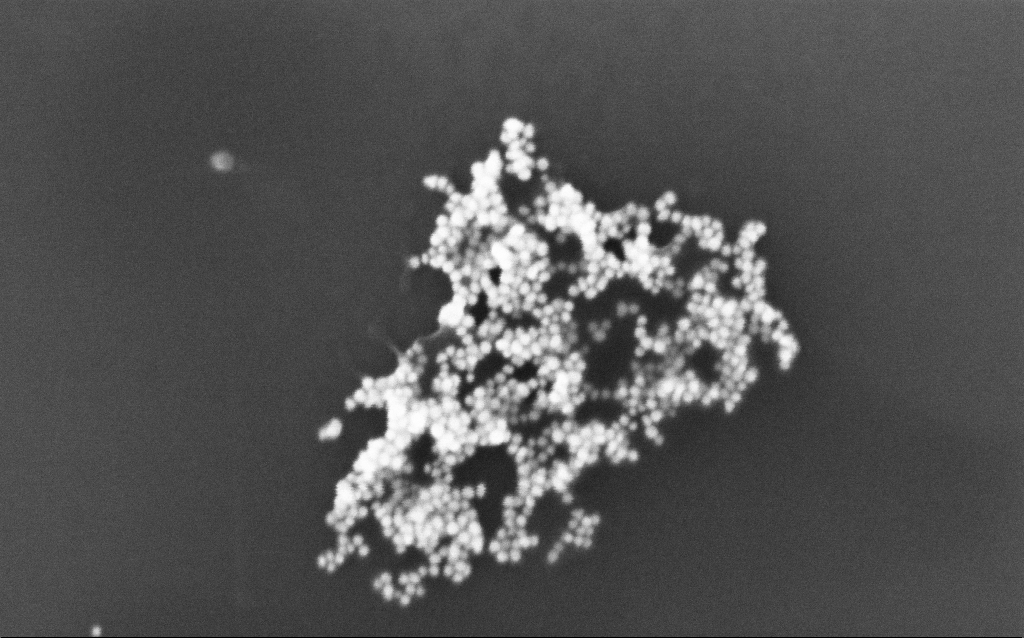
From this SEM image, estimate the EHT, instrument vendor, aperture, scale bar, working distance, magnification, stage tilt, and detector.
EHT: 4 kV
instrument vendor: Zeiss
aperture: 30 µm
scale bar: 200 nm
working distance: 2.9 mm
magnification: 265.76 K X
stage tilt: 0°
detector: InLens